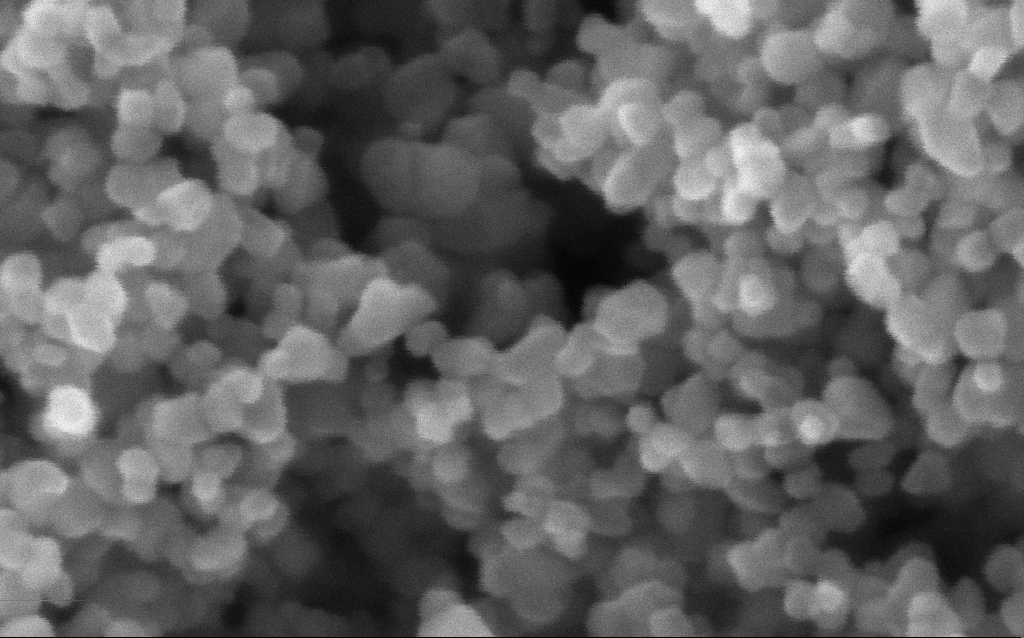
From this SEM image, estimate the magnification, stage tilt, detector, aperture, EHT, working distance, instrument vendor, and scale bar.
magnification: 716 K X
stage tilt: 0°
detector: InLens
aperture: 30 µm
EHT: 5 kV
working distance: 2.9 mm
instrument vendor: Zeiss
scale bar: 100 nm